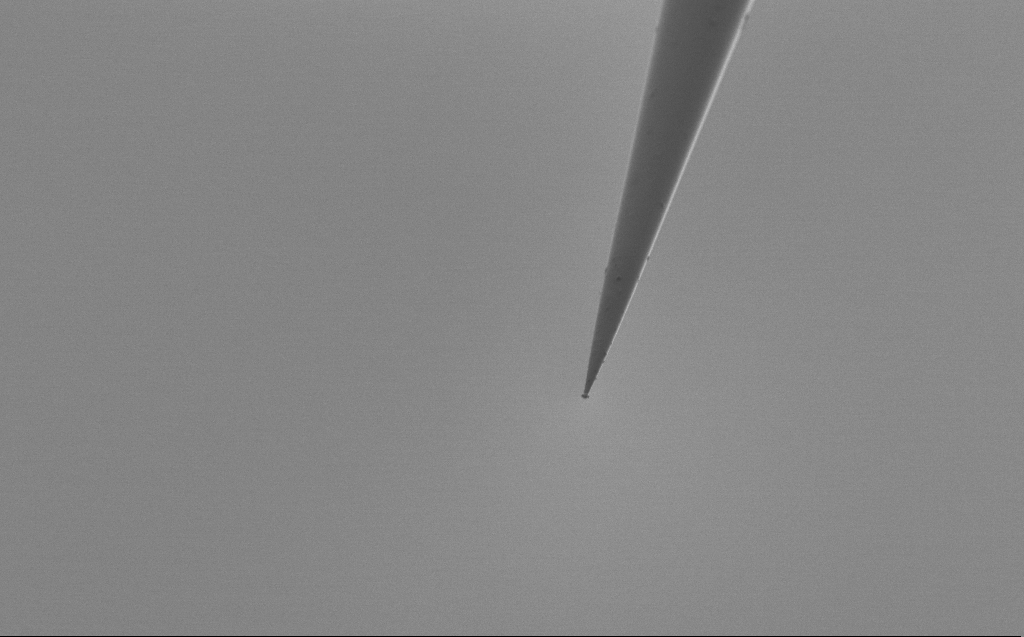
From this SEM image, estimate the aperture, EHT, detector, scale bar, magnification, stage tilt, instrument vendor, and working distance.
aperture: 30 µm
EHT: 1 kV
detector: SE2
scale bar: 10000 nm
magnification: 5 K X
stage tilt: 45°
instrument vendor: Zeiss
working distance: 5 mm